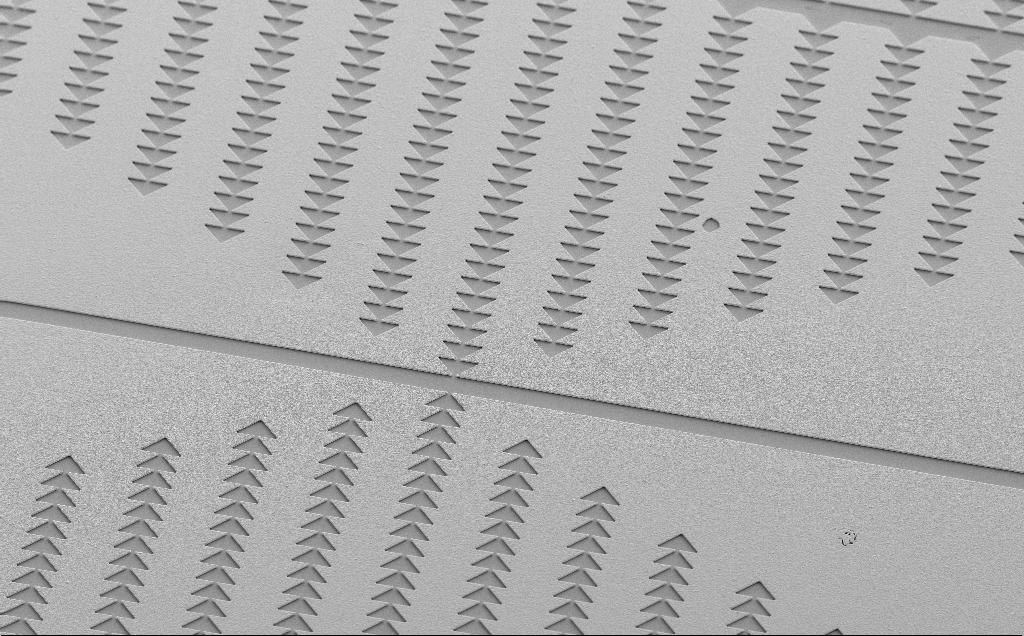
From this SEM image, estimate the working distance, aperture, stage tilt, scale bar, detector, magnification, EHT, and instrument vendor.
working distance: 13 mm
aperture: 30 µm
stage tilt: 35°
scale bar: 100000 nm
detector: SE2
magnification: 0.414 K X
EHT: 5 kV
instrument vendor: Zeiss